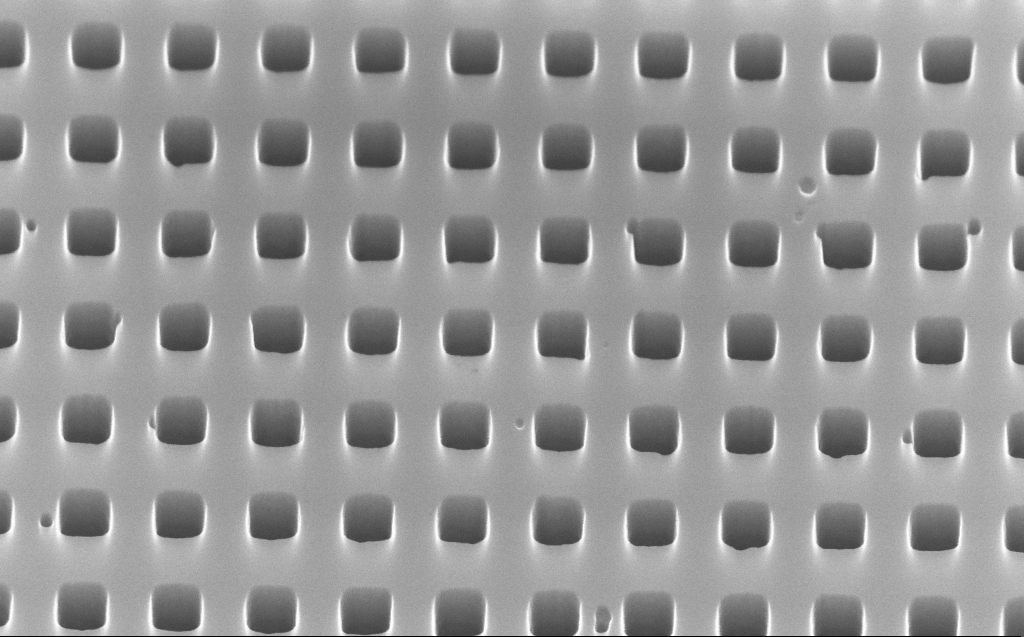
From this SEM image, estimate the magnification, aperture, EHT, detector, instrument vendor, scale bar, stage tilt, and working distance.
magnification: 70.21 K X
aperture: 30 µm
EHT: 10 kV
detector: InLens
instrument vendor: Zeiss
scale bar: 1000 nm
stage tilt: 45°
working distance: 6 mm